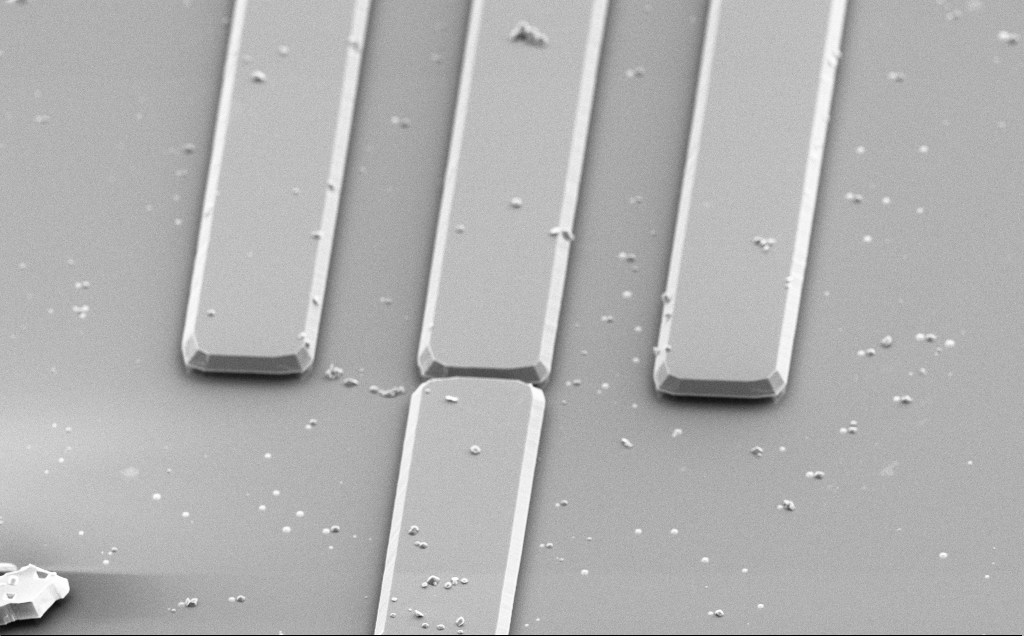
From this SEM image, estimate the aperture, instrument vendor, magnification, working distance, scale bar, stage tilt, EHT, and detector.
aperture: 30 µm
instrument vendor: Zeiss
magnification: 2.19 K X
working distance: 12 mm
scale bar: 20000 nm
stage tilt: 50°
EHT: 5 kV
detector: SE2